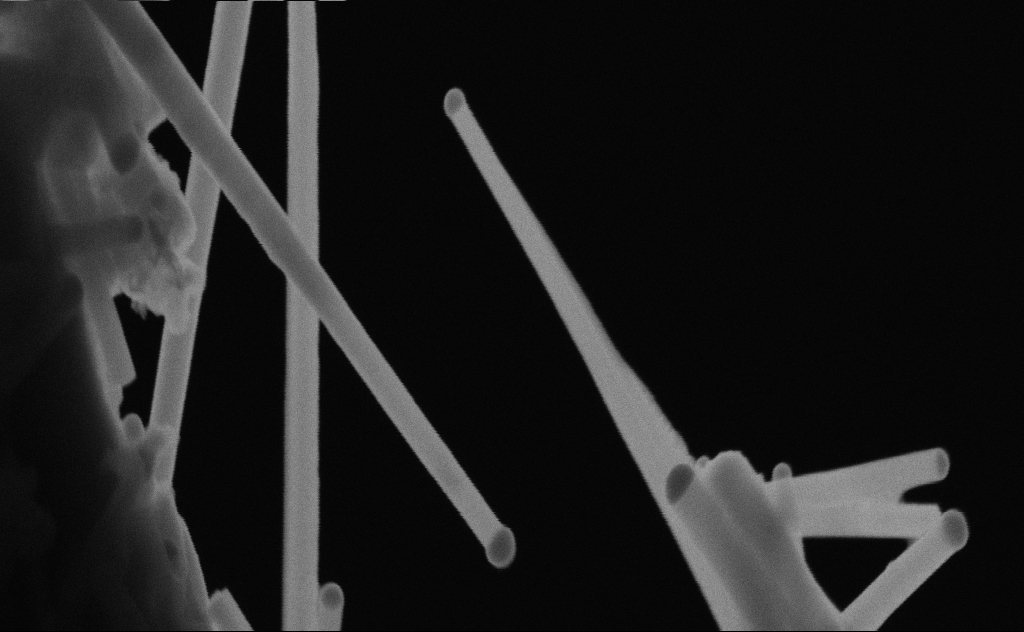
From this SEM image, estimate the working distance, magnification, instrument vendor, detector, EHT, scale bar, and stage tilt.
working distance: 9 mm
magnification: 160.41 K X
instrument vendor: Zeiss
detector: SE2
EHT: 20 kV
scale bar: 200 nm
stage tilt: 0°